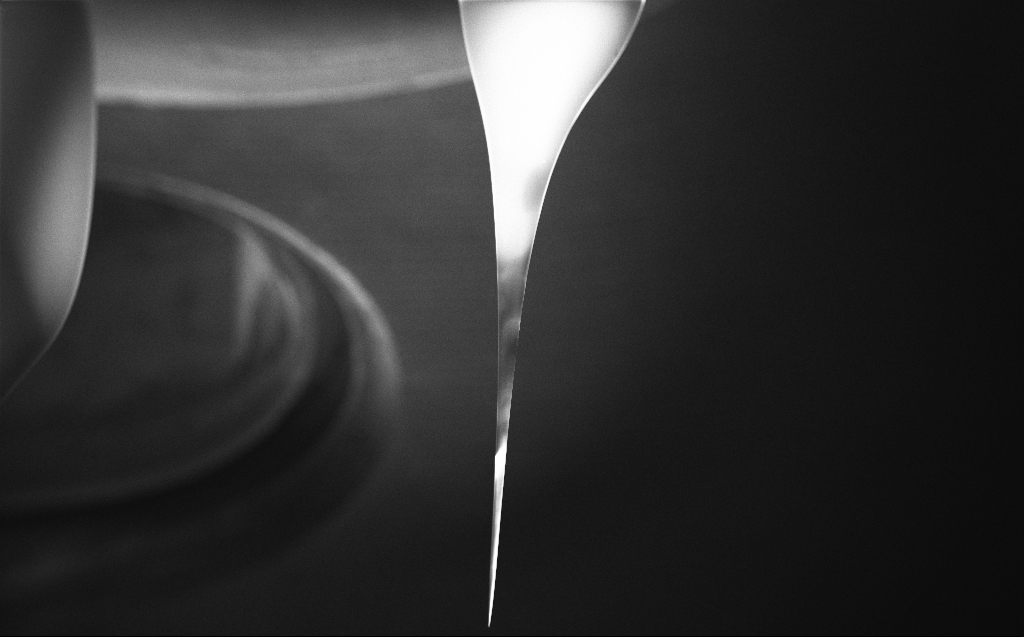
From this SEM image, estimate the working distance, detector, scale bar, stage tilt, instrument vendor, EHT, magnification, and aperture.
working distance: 5 mm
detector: InLens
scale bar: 200000 nm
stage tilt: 45°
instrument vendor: Zeiss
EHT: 5 kV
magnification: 0.083 K X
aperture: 20 µm